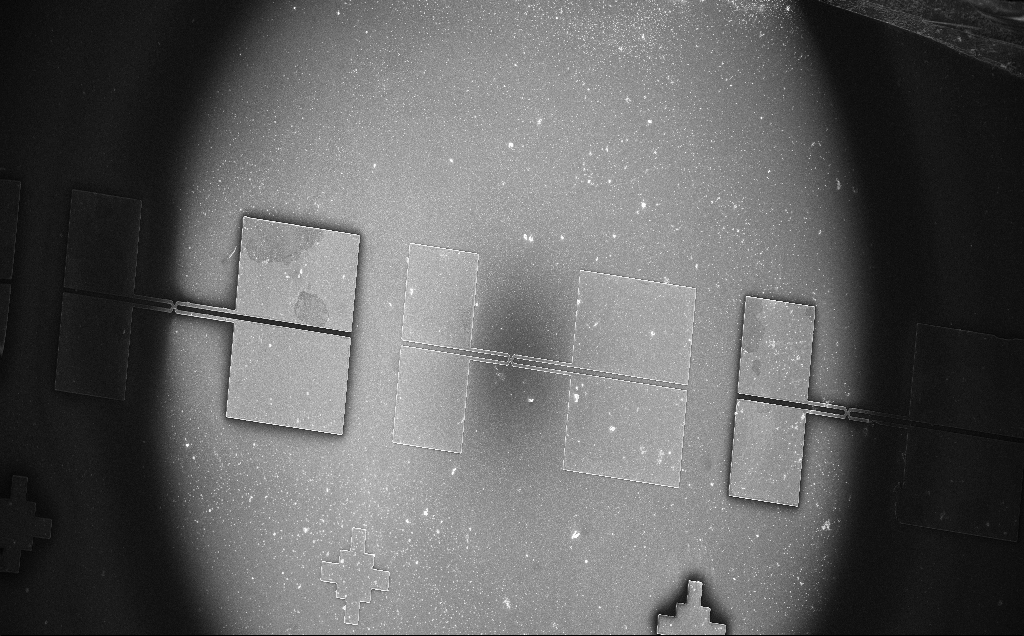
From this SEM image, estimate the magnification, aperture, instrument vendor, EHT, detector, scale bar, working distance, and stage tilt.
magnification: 0.125 K X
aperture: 30 µm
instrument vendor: Zeiss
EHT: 15 kV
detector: InLens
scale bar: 100000 nm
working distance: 4 mm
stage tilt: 0°